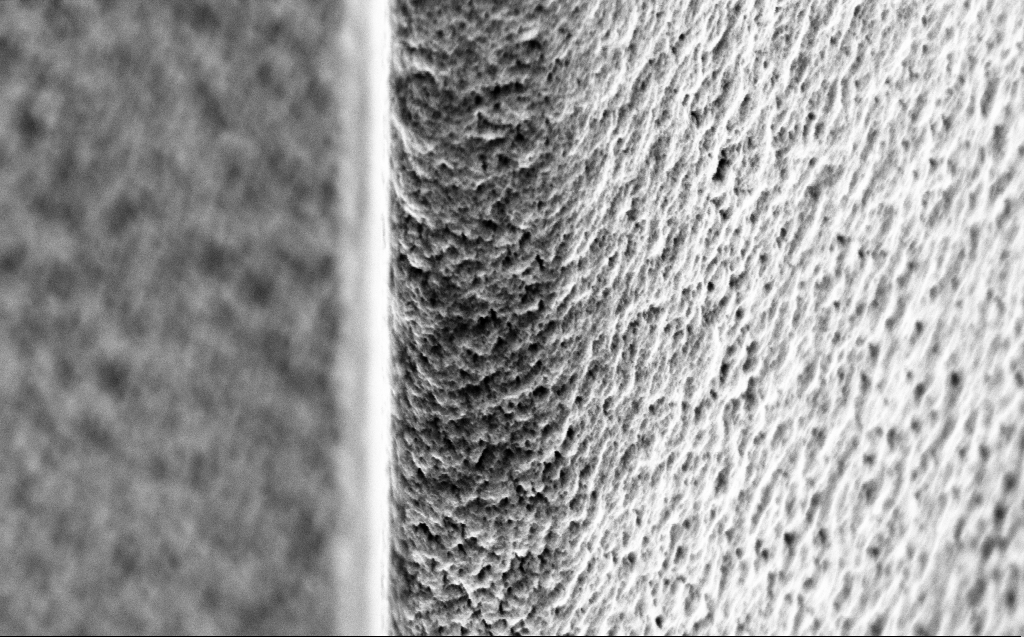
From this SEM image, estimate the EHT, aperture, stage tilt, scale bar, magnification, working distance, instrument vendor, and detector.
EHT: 5 kV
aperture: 30 µm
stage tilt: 45°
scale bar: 1000 nm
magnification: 64.38 K X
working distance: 5 mm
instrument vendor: Zeiss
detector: InLens